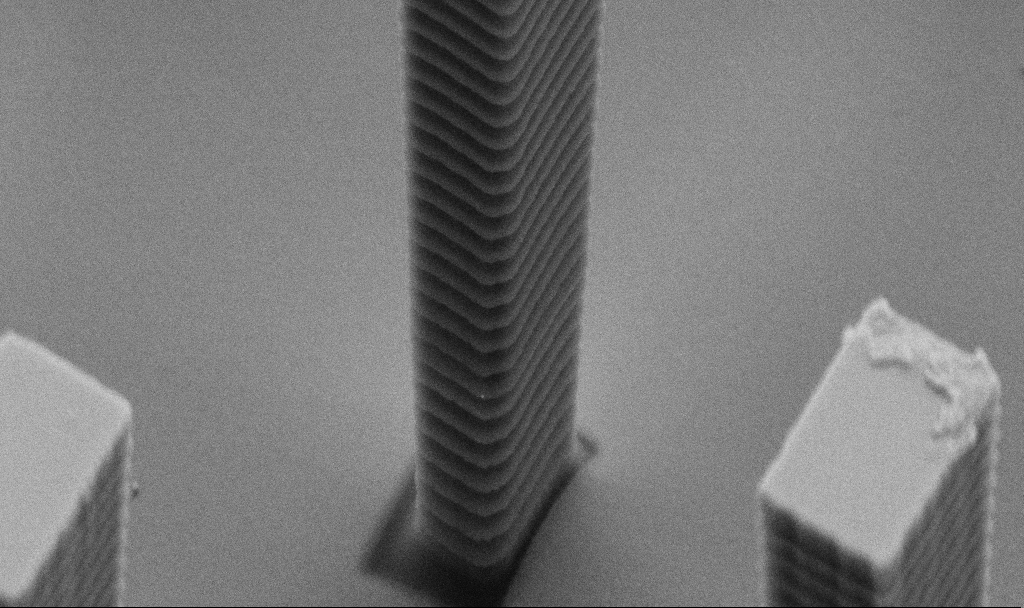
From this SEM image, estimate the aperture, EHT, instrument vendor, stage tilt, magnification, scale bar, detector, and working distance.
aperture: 30 µm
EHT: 5 kV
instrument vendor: Zeiss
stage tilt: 44.9°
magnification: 29.41 K X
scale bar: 1000 nm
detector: SE2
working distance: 5.7 mm